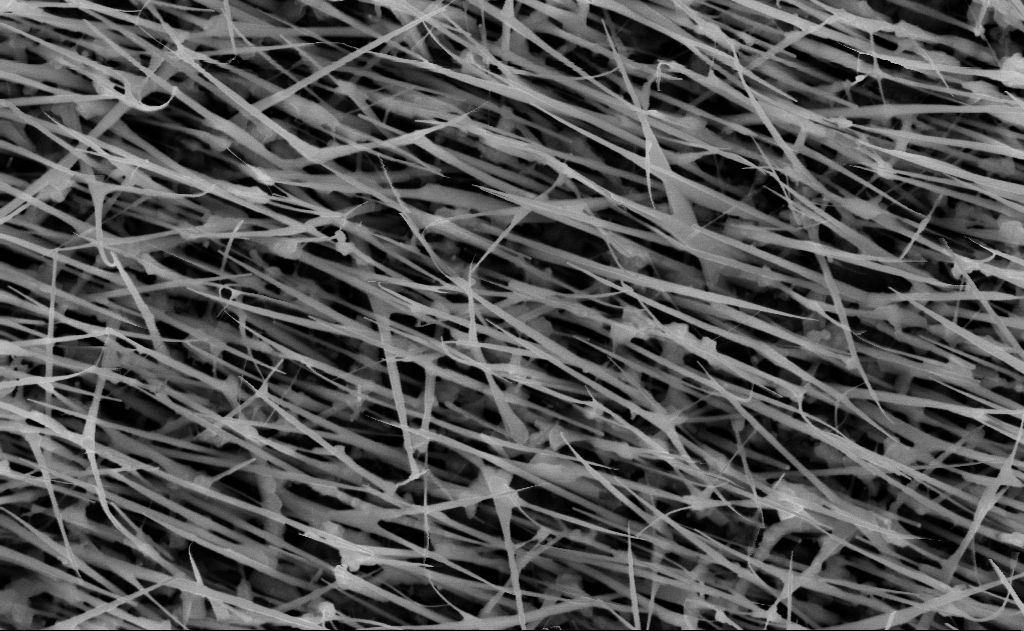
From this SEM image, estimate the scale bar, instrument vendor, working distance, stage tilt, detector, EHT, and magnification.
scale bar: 1000 nm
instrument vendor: Zeiss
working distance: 15 mm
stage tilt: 0°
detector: InLens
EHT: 10 kV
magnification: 40 K X